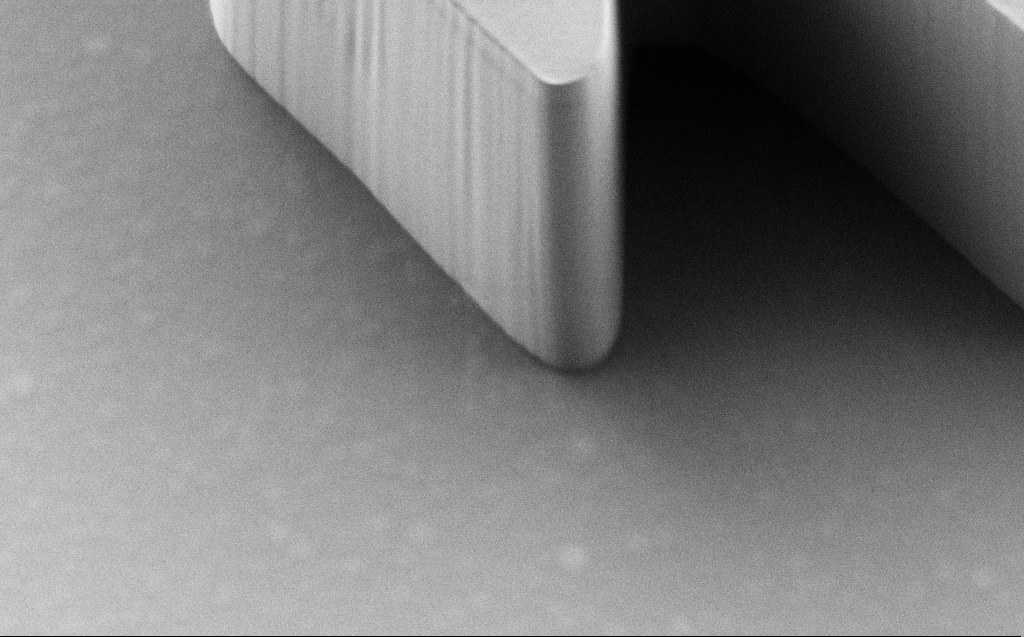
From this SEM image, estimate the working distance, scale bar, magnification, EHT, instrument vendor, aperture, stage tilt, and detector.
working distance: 7 mm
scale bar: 2000 nm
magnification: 16.58 K X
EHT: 1 kV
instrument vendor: Zeiss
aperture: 30 µm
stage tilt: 30°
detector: SE2